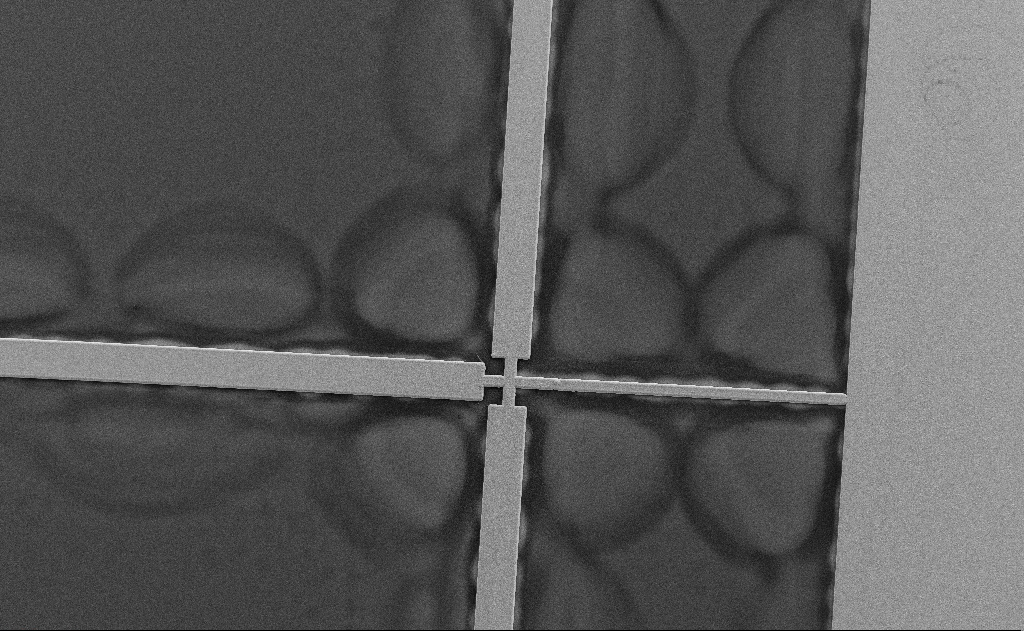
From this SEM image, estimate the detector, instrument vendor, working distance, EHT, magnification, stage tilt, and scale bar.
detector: SE2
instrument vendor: Zeiss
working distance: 6 mm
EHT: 10 kV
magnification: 0.247 K X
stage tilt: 0°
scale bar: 100000 nm